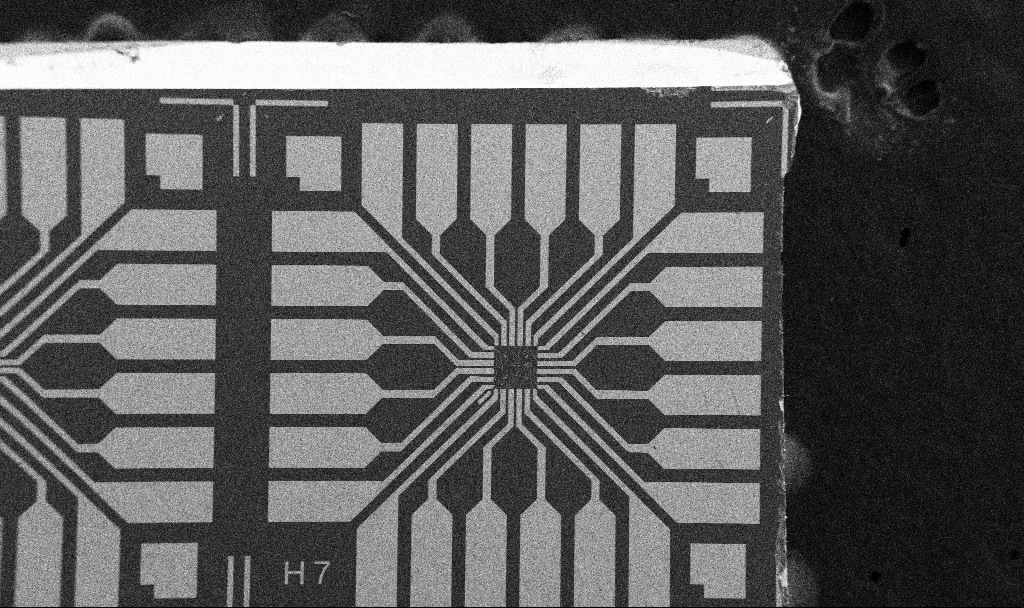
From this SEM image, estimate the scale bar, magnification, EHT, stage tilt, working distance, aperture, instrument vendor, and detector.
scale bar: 200000 nm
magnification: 0.1 K X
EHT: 5 kV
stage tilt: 0°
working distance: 10.7 mm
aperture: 30 µm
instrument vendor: Zeiss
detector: SE2